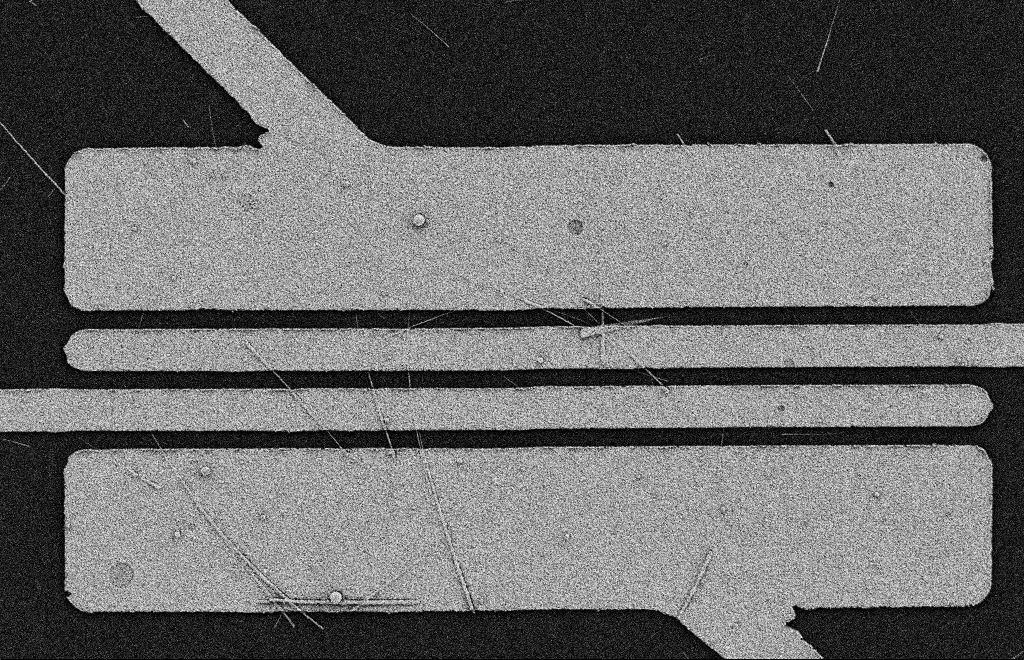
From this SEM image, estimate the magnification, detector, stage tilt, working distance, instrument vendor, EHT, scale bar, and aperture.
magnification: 5.5 K X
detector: SE2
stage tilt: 0°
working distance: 11 mm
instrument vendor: Zeiss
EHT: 2 kV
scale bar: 2000 nm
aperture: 20 µm